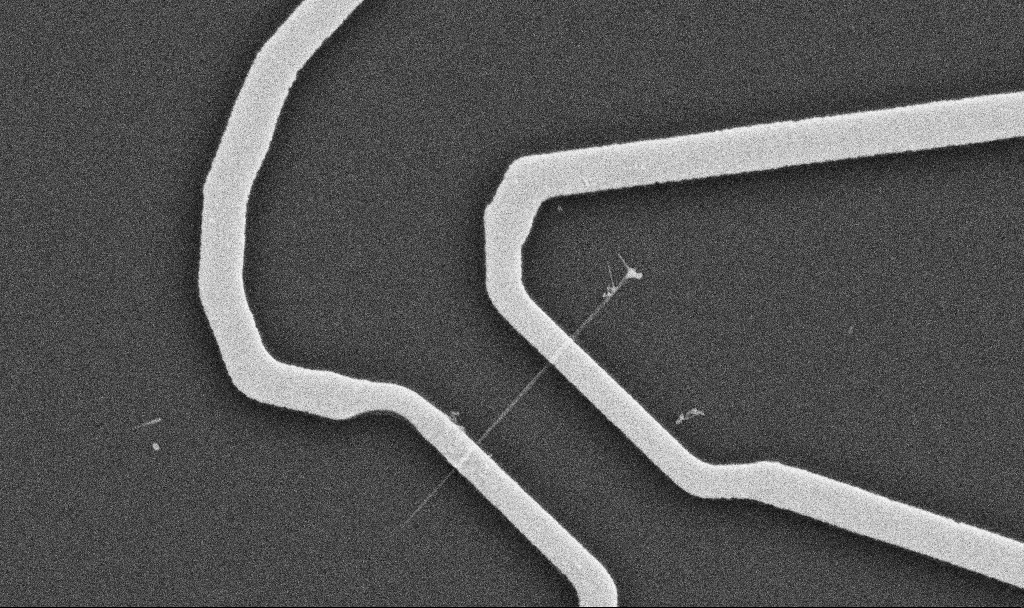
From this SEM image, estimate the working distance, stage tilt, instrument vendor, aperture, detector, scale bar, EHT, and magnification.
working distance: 10.7 mm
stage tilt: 0°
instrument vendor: Zeiss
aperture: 30 µm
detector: SE2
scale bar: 1000 nm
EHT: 10 kV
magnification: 20 K X